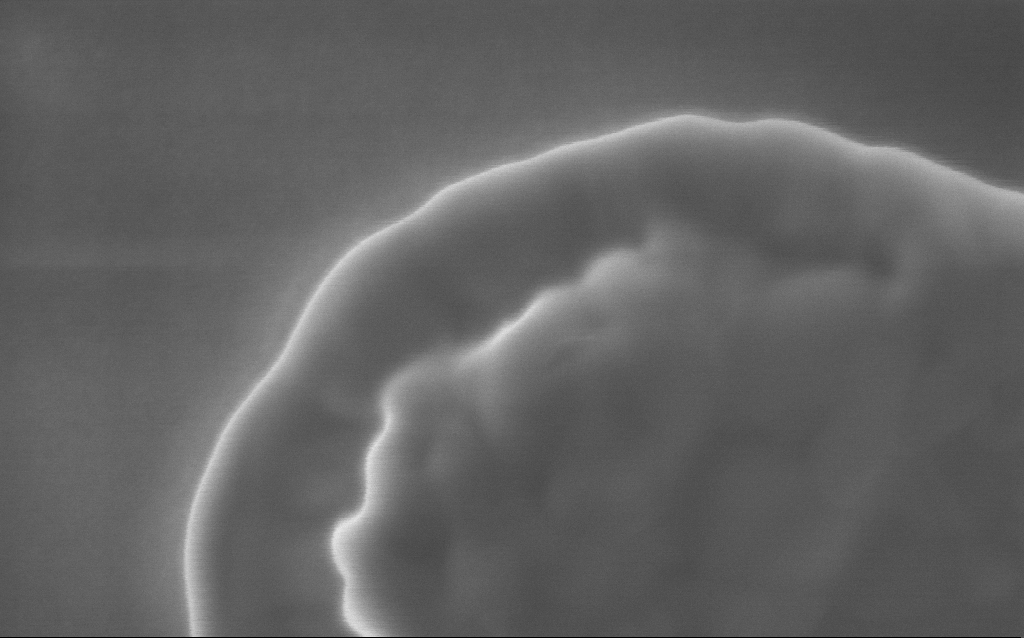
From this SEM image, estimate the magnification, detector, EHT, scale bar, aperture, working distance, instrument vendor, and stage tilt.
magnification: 217 K X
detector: InLens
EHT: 5 kV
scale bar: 200 nm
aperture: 30 µm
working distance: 3 mm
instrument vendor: Zeiss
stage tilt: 0°